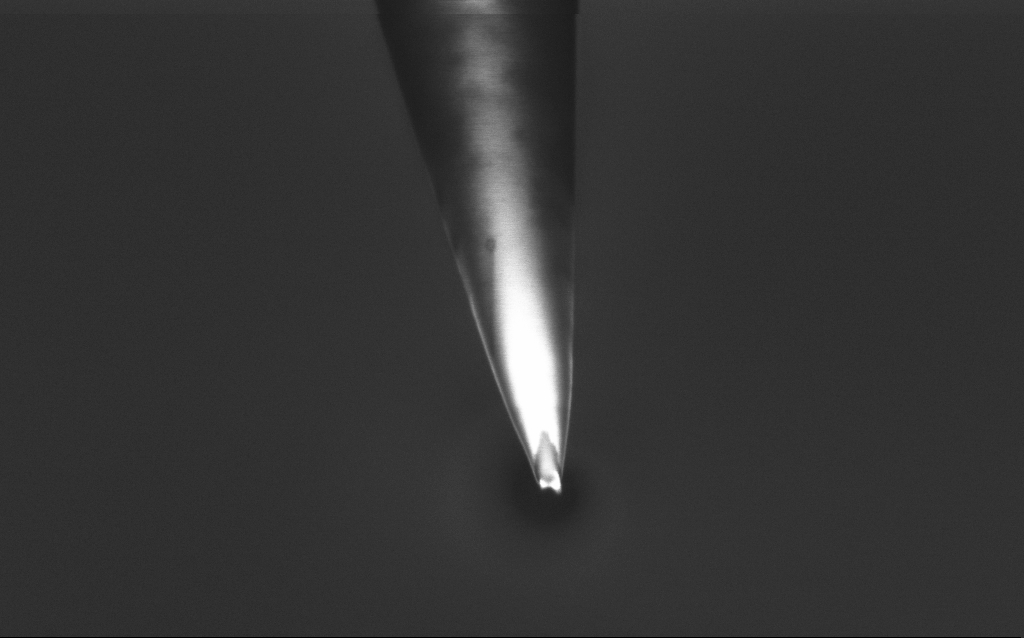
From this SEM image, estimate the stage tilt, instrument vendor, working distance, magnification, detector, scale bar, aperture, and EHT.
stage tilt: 45°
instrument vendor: Zeiss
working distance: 6 mm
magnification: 50 K X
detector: InLens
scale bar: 1000 nm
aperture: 30 µm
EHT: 1 kV